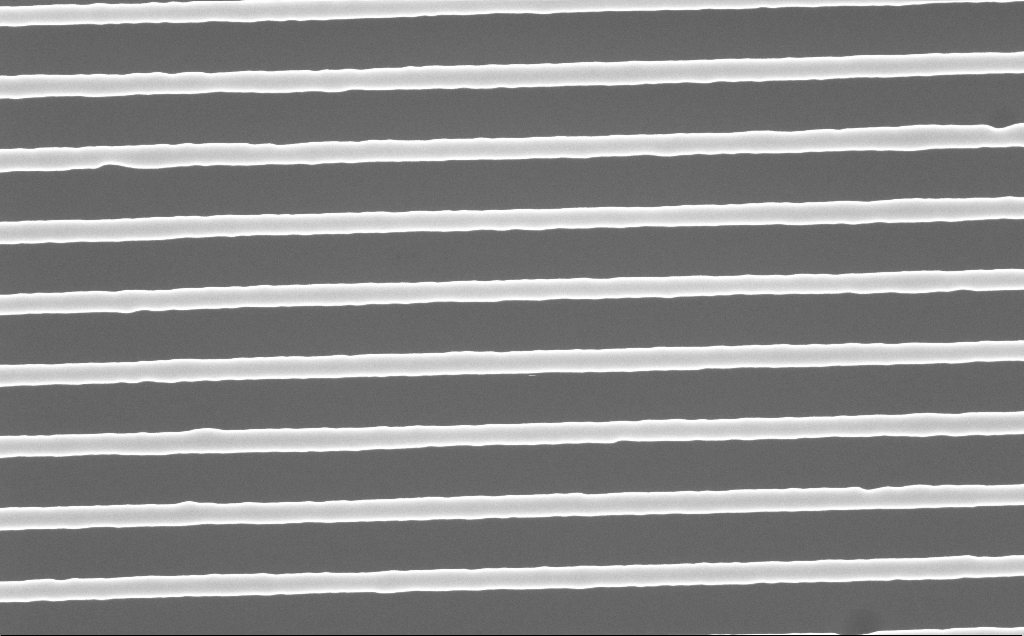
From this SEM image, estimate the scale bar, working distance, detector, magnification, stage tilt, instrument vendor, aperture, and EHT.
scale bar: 200 nm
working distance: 4 mm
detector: InLens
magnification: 106.33 K X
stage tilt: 0°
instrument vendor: Zeiss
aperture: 30 µm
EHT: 10 kV